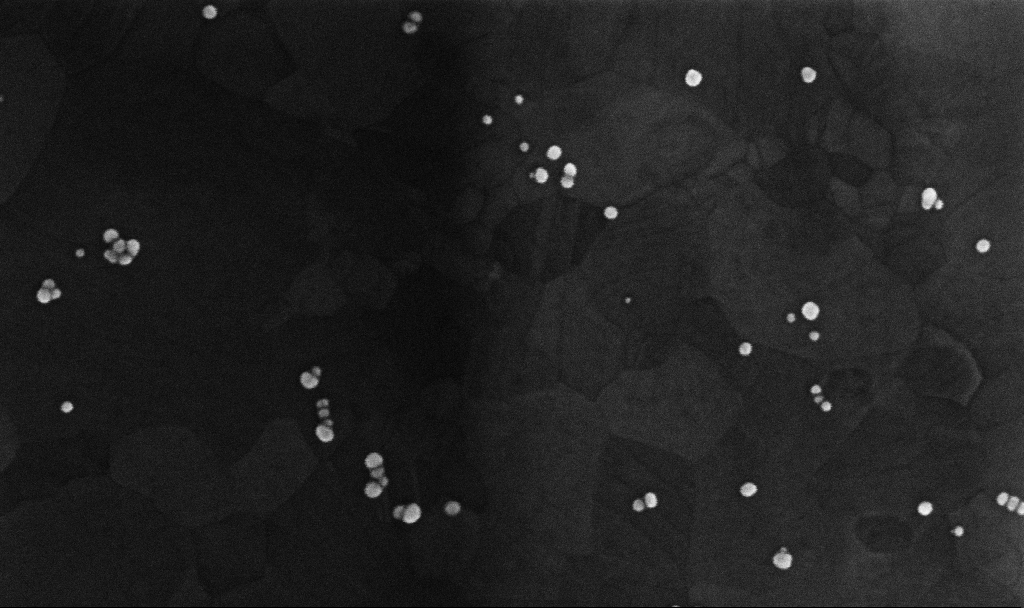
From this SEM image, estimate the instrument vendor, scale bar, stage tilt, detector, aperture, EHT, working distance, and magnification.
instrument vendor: Zeiss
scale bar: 200 nm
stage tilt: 0°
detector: InLens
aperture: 30 µm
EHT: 10 kV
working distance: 3.4 mm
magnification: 229.63 K X